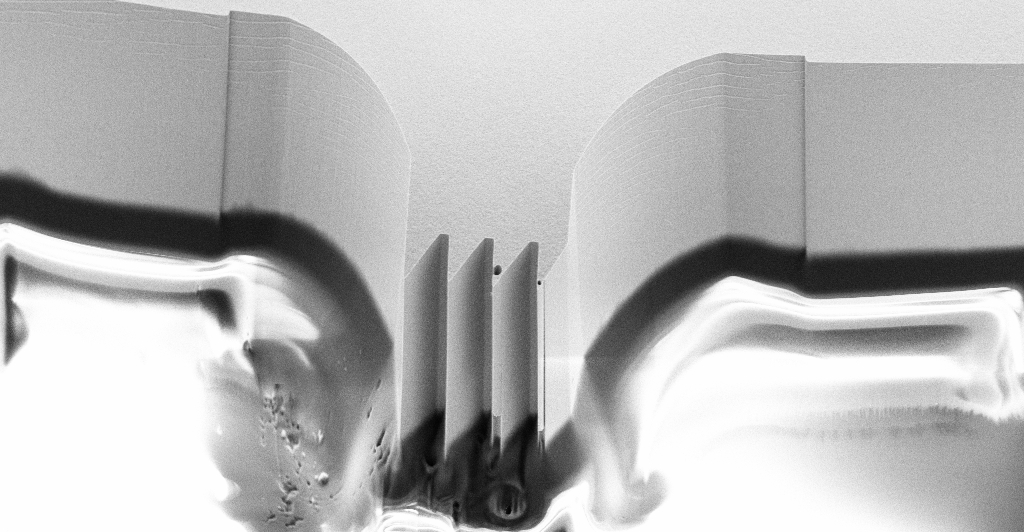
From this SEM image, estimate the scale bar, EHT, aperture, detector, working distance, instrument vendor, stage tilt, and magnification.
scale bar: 20000 nm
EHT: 10 kV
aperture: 30 µm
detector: SE2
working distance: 7 mm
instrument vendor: Zeiss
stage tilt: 45°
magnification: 1.44 K X